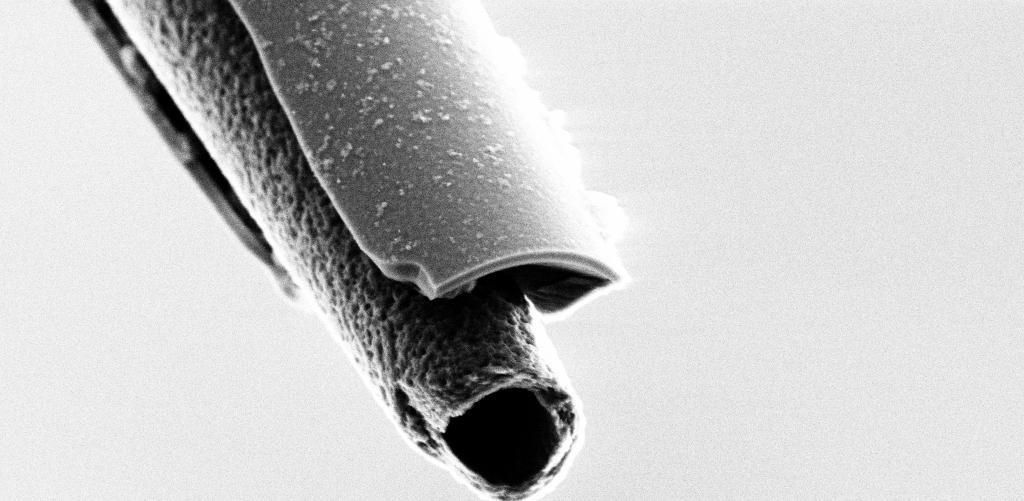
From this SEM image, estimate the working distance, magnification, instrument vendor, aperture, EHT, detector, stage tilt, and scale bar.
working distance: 7.4 mm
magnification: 25 K X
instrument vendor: Zeiss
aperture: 30 µm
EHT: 3 kV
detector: SE2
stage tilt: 45°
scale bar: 2000 nm